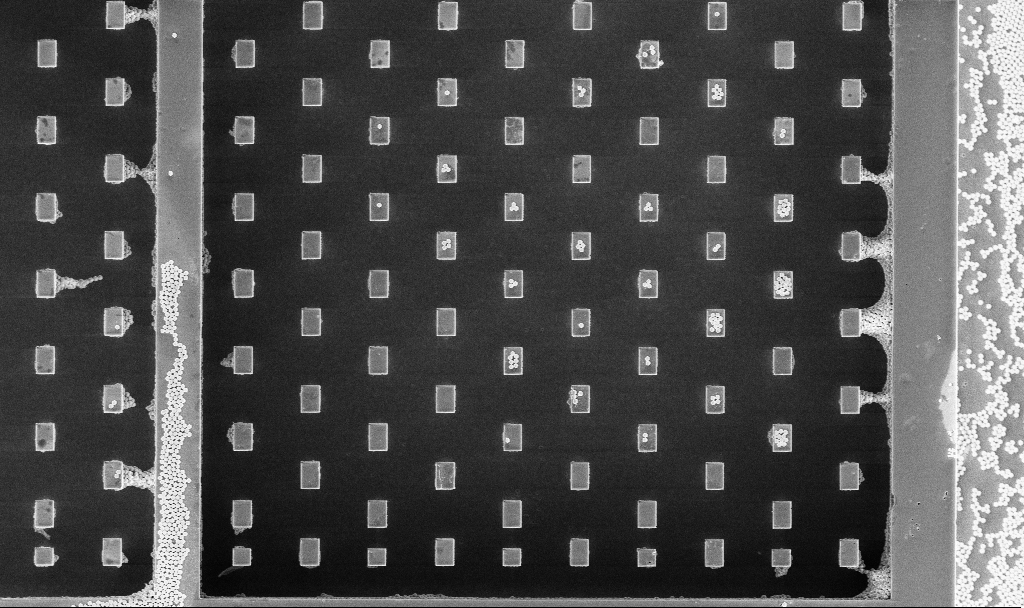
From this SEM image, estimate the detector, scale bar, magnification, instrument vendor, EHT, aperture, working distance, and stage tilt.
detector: InLens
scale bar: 10000 nm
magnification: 2.37 K X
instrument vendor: Zeiss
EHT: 5 kV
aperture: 30 µm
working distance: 3.2 mm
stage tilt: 0°